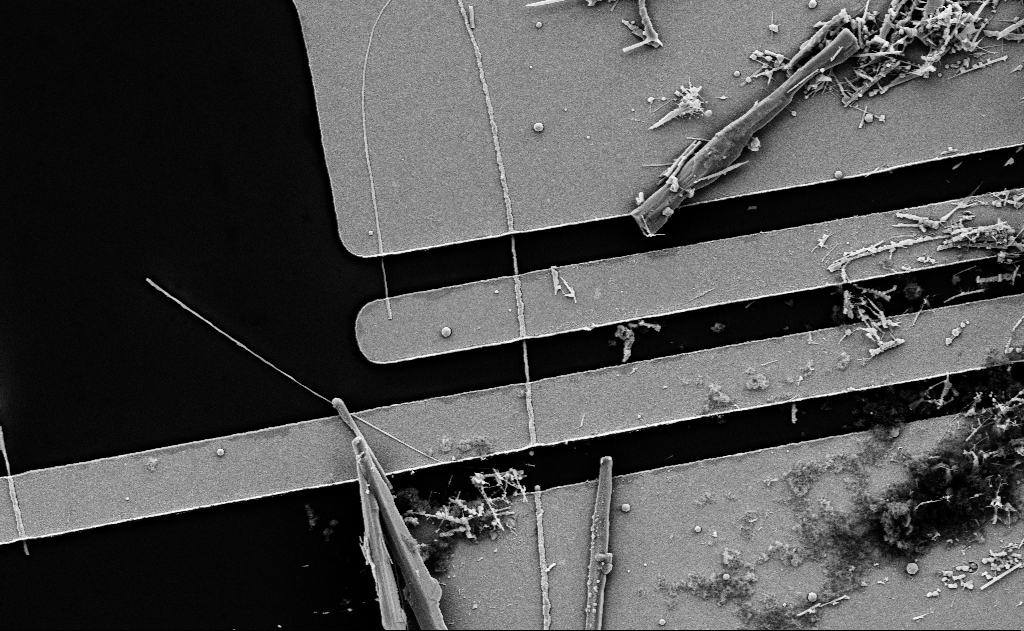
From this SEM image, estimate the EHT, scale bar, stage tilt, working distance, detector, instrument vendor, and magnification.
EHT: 5 kV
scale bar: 2000 nm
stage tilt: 0°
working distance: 18 mm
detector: SE2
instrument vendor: Zeiss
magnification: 10 K X